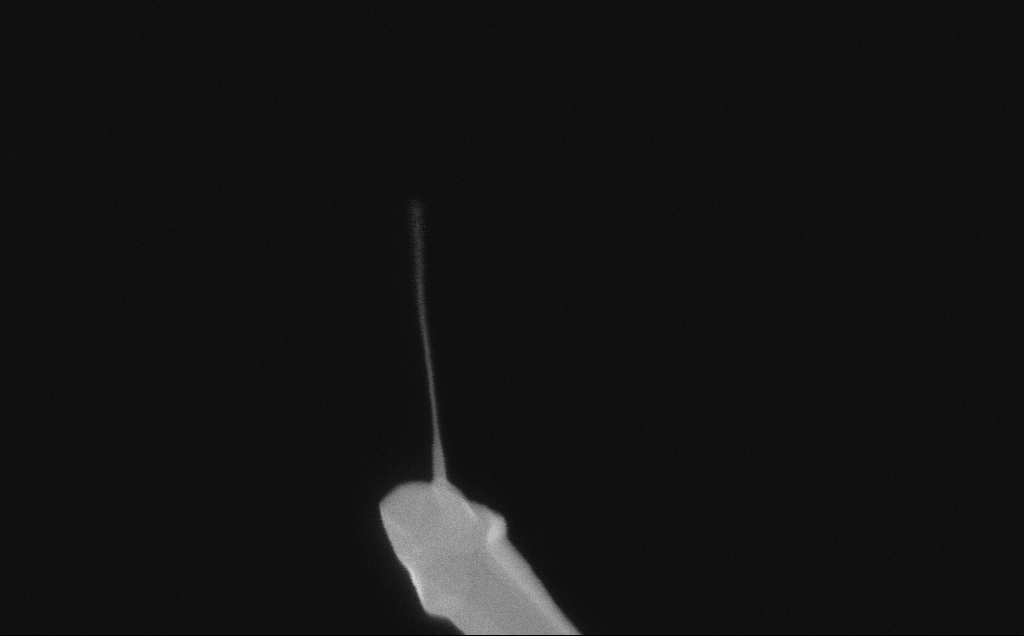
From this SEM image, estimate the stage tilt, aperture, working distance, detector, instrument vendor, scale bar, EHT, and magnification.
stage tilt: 0°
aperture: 30 µm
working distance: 6 mm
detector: InLens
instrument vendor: Zeiss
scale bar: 200 nm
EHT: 10 kV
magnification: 189.64 K X